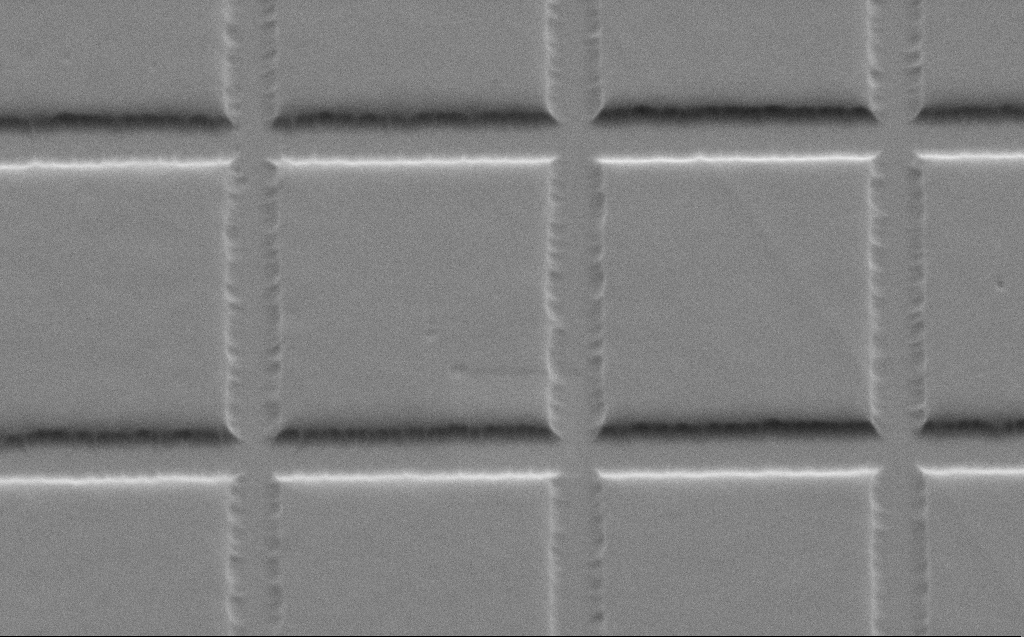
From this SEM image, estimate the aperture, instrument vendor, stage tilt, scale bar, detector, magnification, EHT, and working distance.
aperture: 30 µm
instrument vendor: Zeiss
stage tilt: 45°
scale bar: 2000 nm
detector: SE2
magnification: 11.91 K X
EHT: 3 kV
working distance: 5 mm